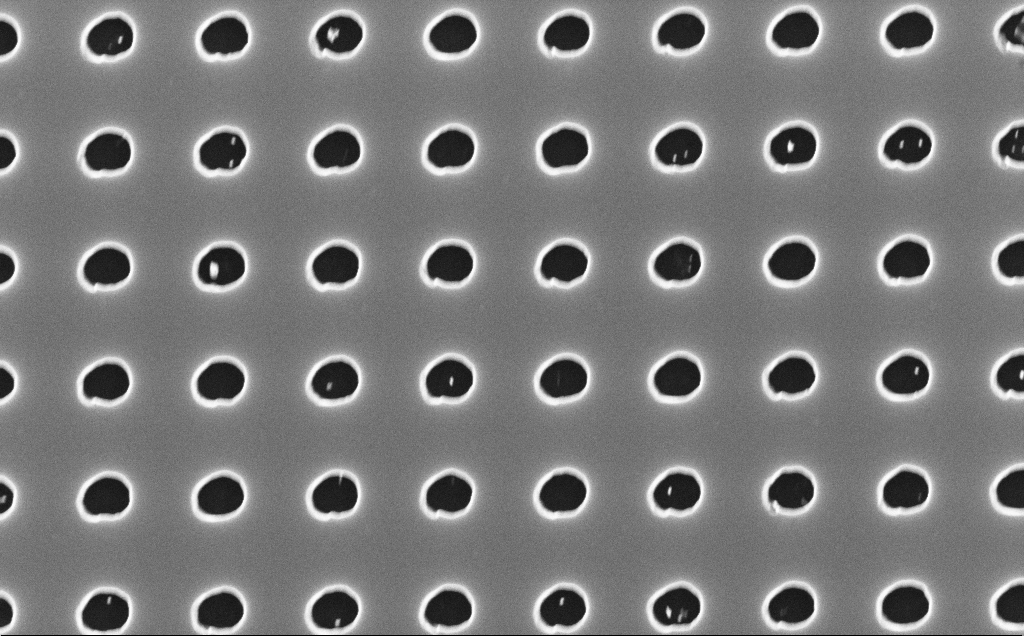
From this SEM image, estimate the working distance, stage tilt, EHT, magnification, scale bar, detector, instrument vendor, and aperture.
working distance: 4 mm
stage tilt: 0°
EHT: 10 kV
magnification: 40 K X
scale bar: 1000 nm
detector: InLens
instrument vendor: Zeiss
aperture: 30 µm